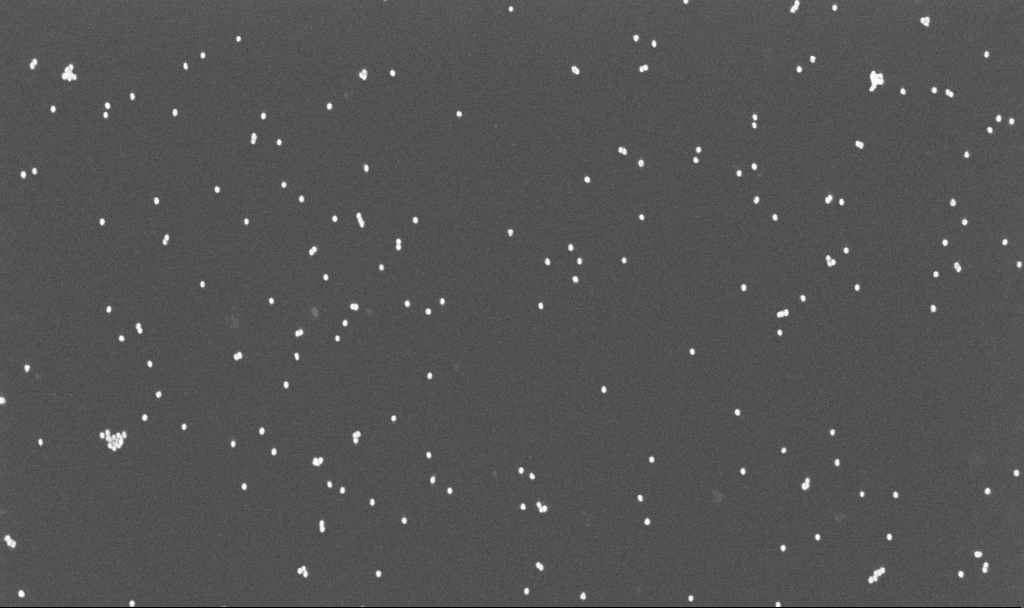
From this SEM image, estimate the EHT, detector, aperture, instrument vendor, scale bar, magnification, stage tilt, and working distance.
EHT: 10 kV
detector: InLens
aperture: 30 µm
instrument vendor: Zeiss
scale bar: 1000 nm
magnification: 70 K X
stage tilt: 0°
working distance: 3.3 mm